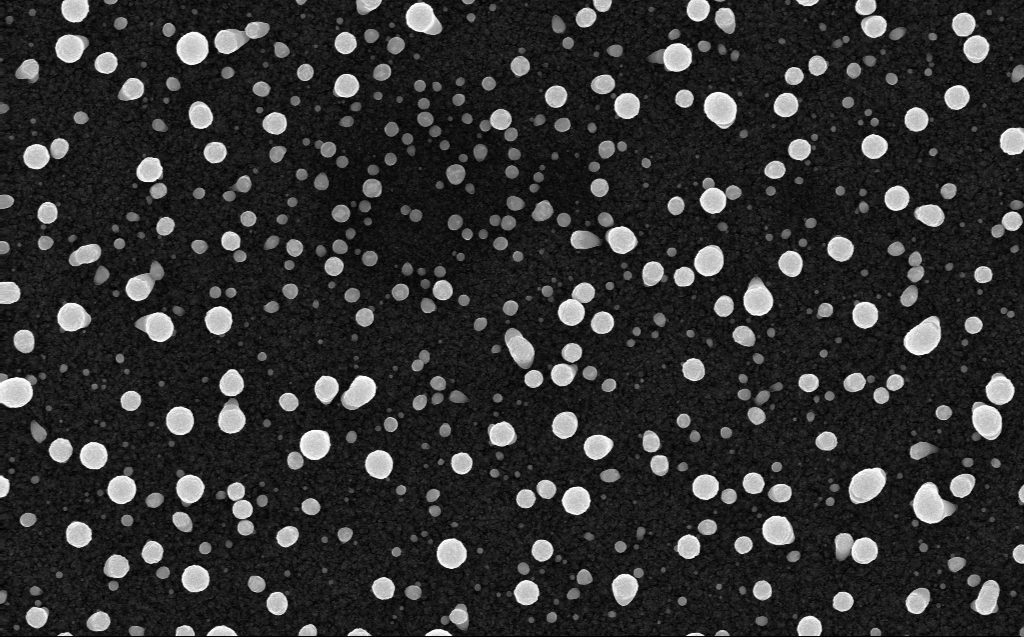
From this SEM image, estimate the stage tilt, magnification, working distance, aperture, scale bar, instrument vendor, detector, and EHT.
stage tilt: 0°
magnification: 50 K X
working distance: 3 mm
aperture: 30 µm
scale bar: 1000 nm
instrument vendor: Zeiss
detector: InLens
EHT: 10 kV